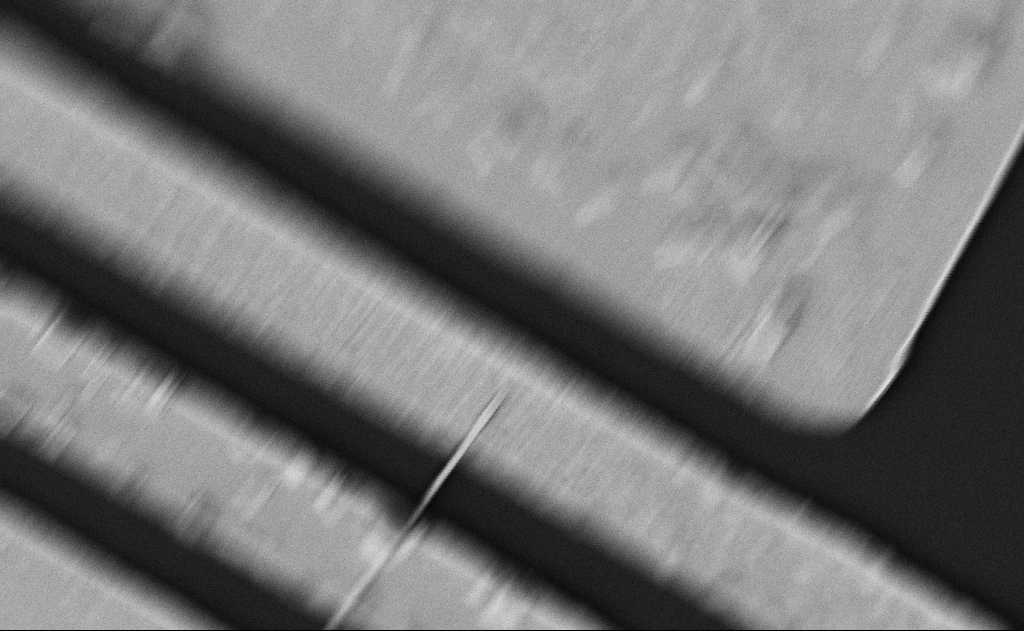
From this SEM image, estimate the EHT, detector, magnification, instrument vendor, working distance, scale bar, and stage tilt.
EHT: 5 kV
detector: SE2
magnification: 18.83 K X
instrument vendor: Zeiss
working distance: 21 mm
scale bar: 1000 nm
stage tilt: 0°